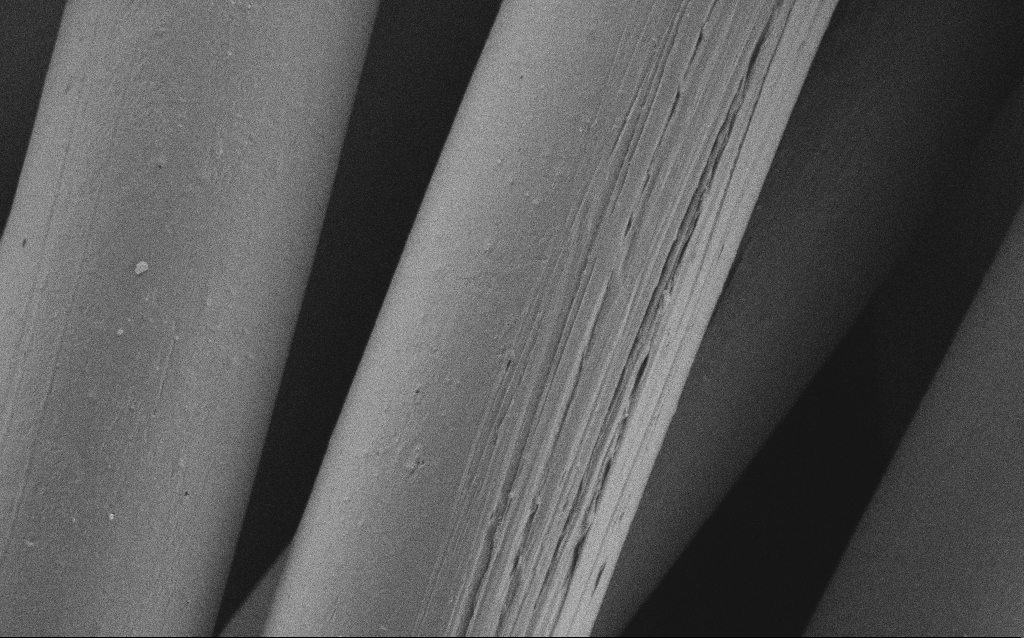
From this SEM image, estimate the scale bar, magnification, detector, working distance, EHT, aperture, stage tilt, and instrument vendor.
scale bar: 10000 nm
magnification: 4.81 K X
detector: SE2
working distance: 4 mm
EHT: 1 kV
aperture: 30 µm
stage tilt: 0°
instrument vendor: Zeiss